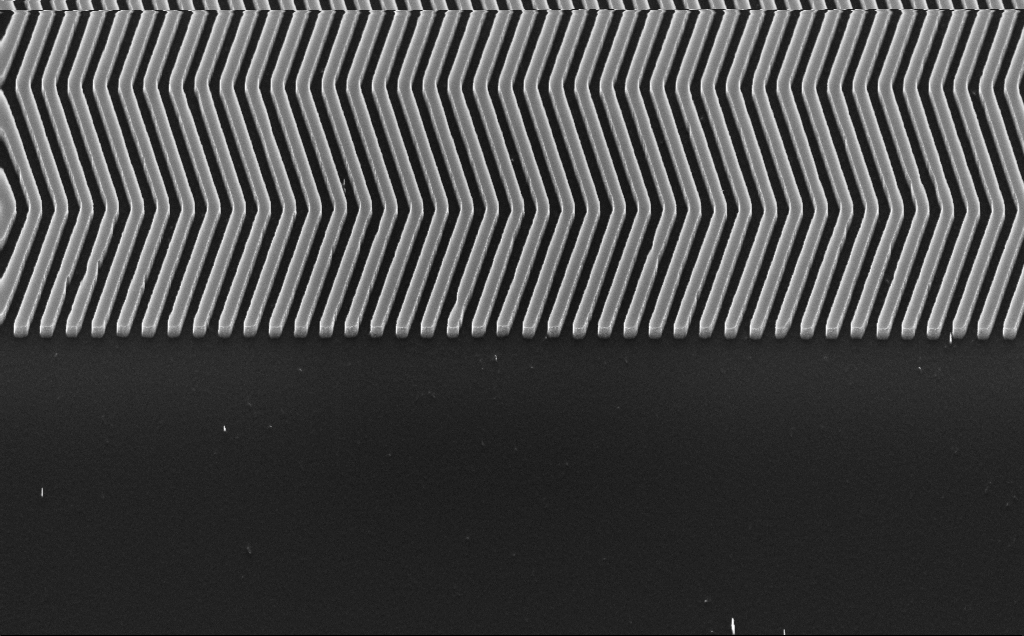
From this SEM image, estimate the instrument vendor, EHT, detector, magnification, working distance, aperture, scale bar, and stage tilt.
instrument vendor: Zeiss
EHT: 10 kV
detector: InLens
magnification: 26.74 K X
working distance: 5 mm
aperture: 30 µm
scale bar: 1000 nm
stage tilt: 30°